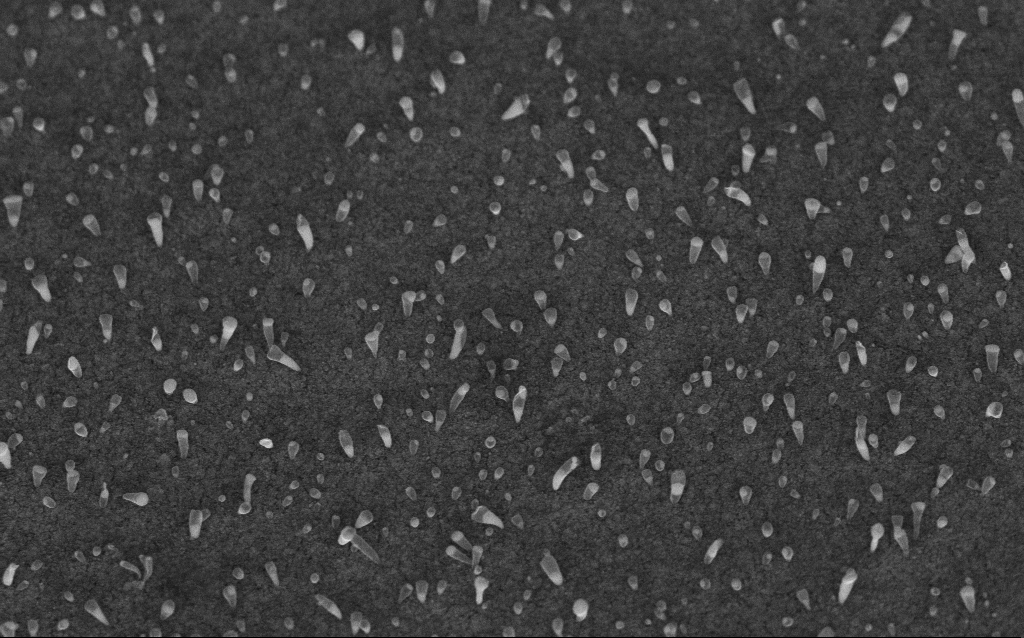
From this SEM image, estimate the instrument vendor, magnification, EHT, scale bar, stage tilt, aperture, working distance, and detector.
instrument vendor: Zeiss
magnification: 50 K X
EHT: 5 kV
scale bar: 1000 nm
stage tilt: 45°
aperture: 30 µm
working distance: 6.7 mm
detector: InLens